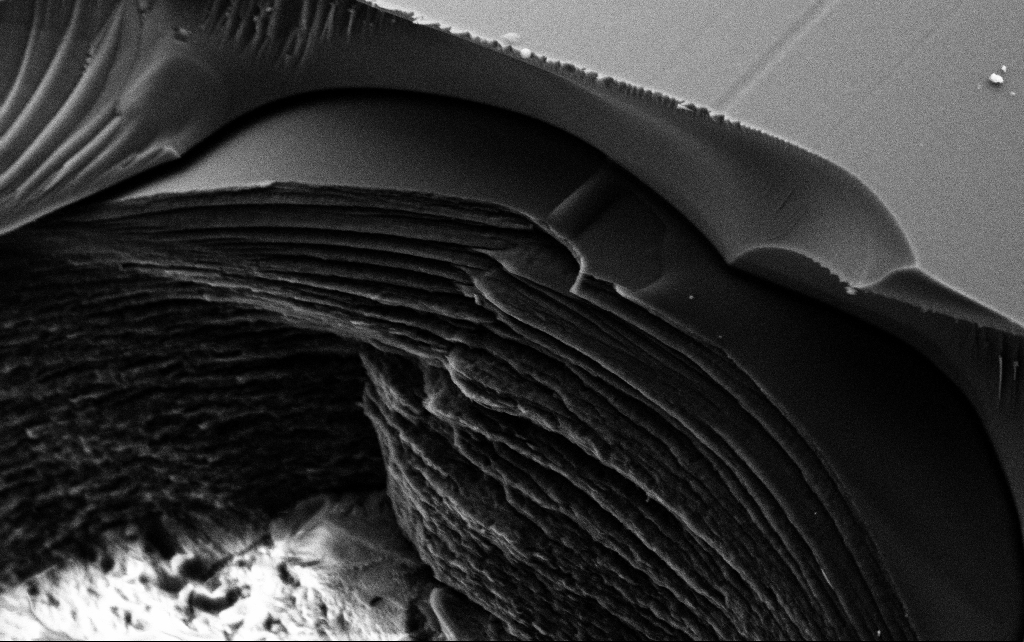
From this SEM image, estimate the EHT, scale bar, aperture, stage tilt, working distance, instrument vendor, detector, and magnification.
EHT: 3 kV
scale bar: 2000 nm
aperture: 30 µm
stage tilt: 45°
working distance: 7.8 mm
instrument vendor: Zeiss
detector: SE2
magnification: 25 K X